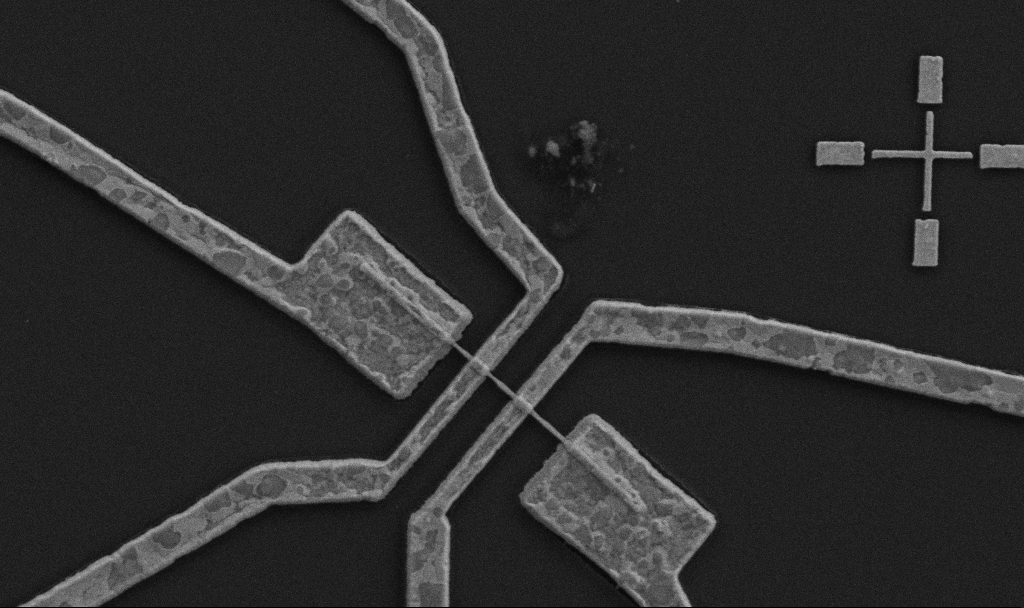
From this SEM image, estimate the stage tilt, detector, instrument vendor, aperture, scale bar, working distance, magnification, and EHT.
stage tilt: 0°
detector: SE2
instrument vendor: Zeiss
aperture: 30 µm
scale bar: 1000 nm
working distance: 10.7 mm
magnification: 20 K X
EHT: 5 kV